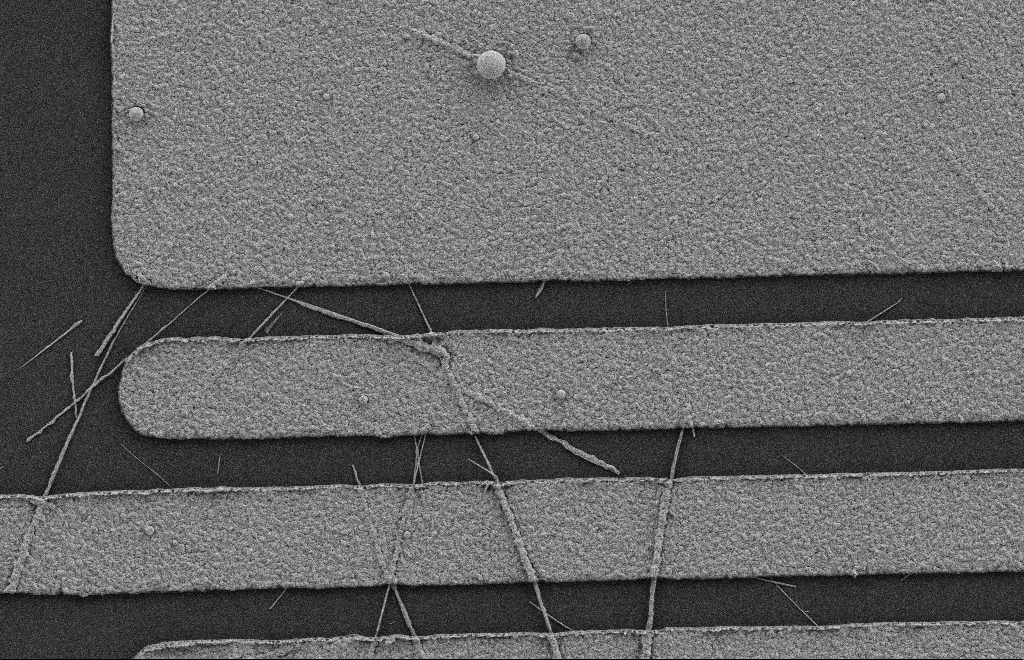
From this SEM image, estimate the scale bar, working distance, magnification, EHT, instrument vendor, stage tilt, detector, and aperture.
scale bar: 2000 nm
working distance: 8 mm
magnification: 13.78 K X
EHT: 2 kV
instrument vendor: Zeiss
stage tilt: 0°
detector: SE2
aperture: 20 µm